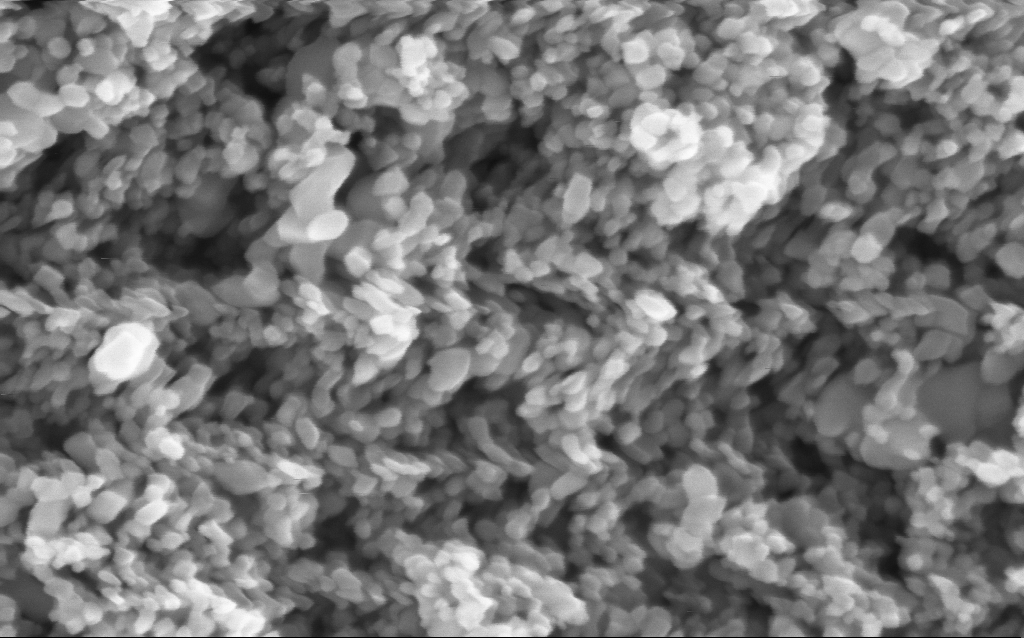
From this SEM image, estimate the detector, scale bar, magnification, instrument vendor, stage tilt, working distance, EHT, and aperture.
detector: InLens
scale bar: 100 nm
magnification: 294.27 K X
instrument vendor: Zeiss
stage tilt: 0°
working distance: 4.5 mm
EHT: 5 kV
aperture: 30 µm